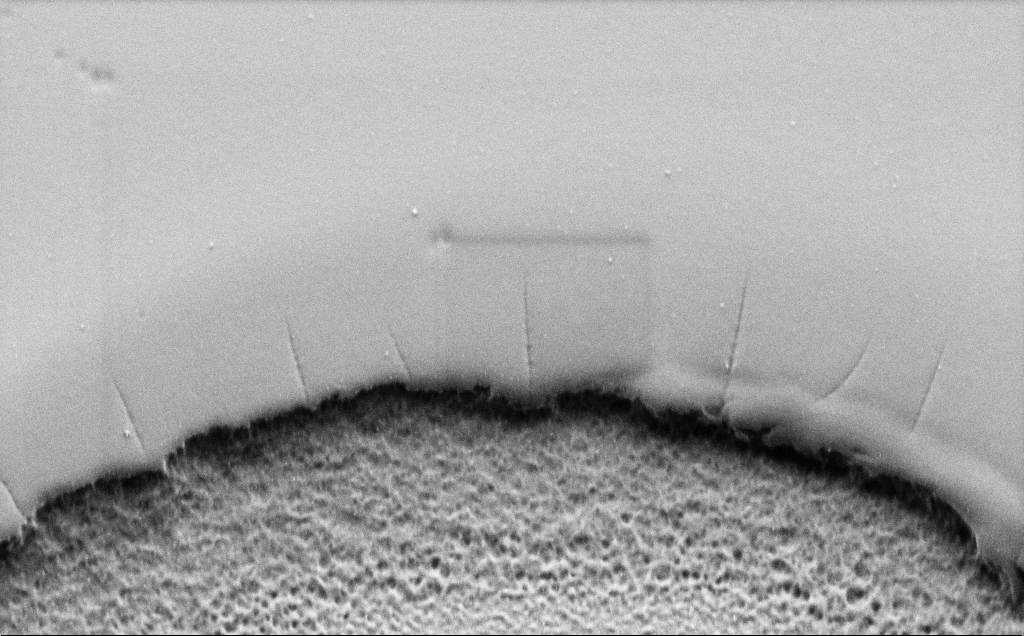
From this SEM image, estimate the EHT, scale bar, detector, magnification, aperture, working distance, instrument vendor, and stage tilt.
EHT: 1.5 kV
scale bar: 2000 nm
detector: SE2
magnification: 26.01 K X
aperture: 30 µm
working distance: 6 mm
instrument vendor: Zeiss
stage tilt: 45°